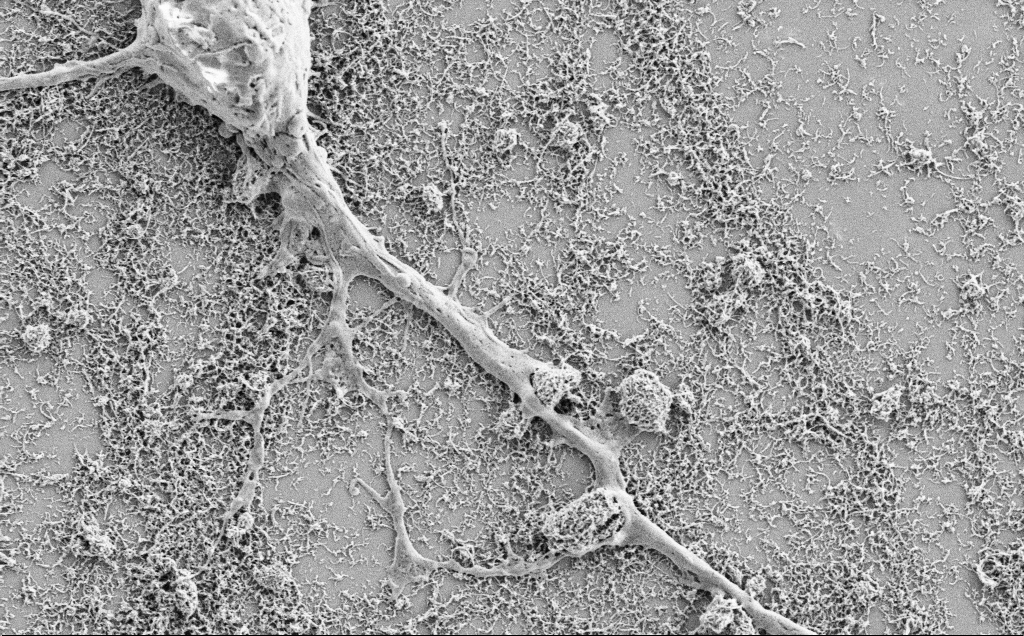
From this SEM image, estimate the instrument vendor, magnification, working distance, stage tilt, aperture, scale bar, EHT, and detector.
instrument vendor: Zeiss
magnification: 10 K X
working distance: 7.1 mm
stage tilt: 0°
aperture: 30 µm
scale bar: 2000 nm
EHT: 2 kV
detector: SE2